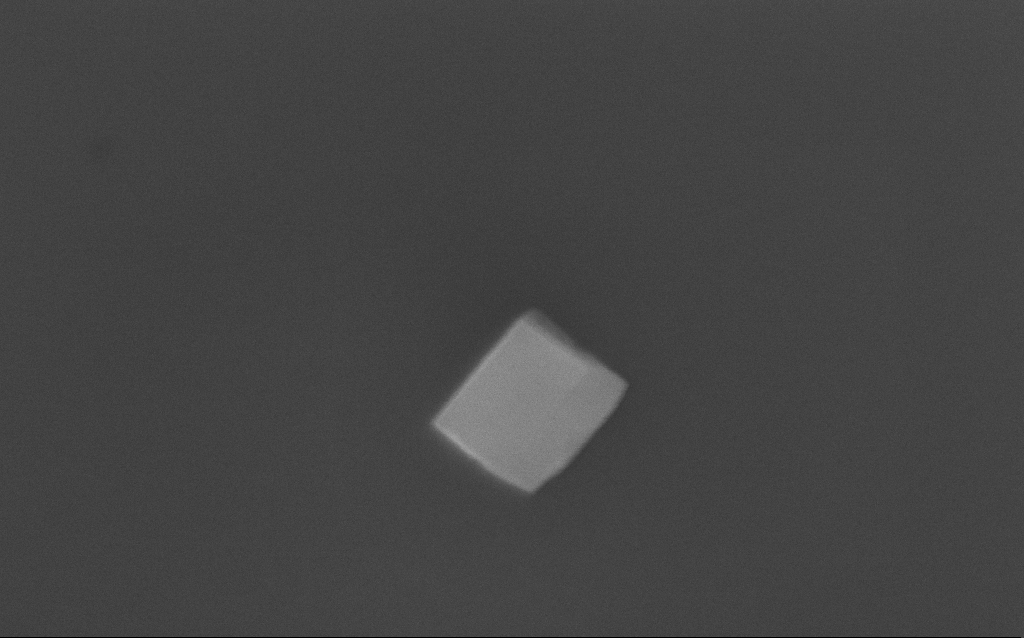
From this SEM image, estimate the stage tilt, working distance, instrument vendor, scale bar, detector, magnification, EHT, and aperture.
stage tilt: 0°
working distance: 3 mm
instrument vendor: Zeiss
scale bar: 200 nm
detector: InLens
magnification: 171.85 K X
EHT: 10 kV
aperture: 30 µm